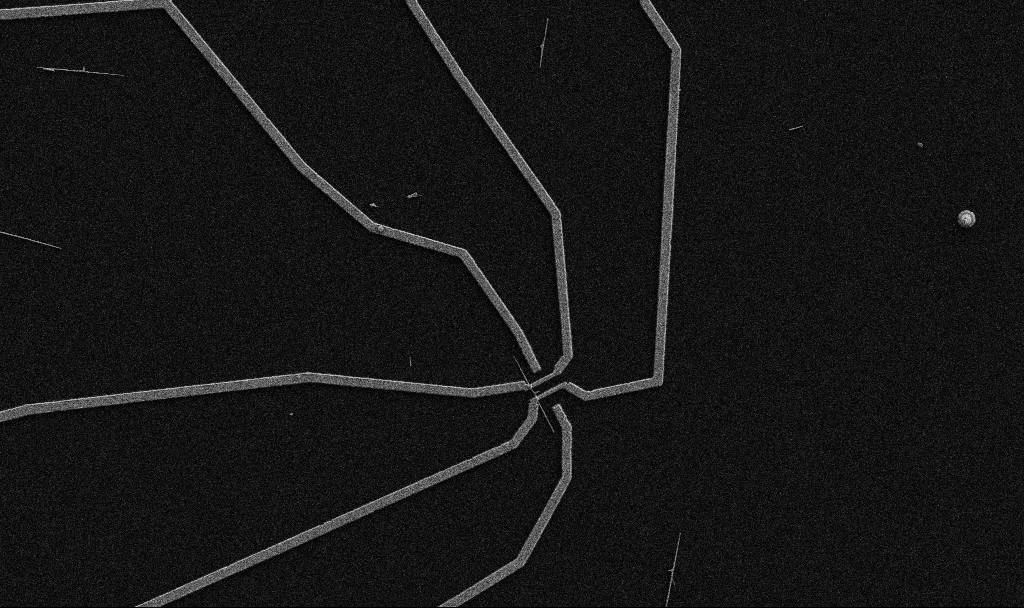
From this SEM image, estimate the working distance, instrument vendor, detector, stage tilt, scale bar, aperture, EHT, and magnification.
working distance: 10.7 mm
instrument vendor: Zeiss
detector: SE2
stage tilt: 0°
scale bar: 10000 nm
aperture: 30 µm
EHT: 5 kV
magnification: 5 K X